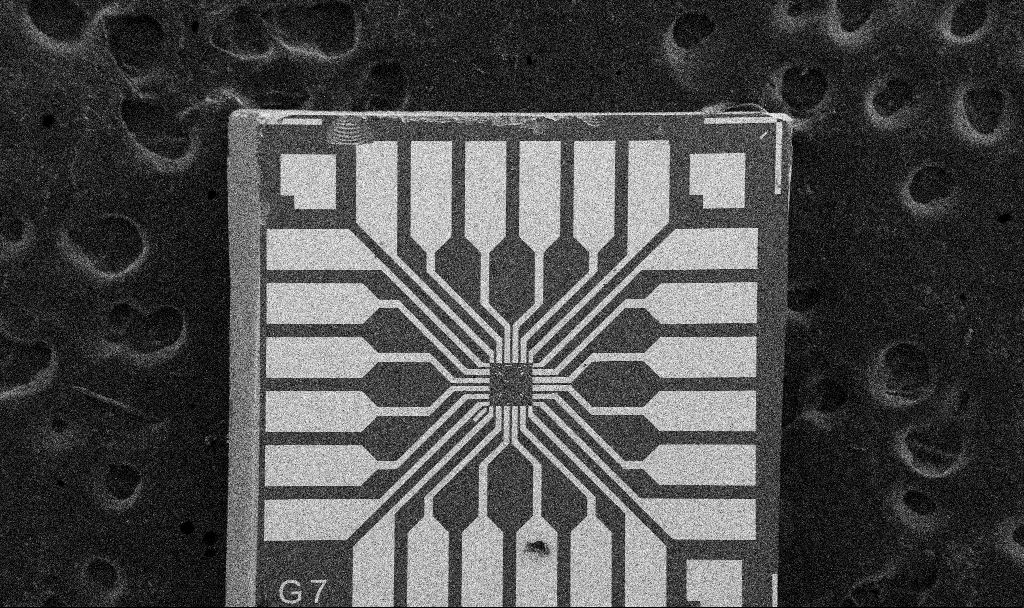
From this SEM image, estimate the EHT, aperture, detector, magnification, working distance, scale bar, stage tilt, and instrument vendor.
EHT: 5 kV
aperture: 30 µm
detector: SE2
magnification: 0.1 K X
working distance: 8.7 mm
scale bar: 200000 nm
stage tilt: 0°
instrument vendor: Zeiss